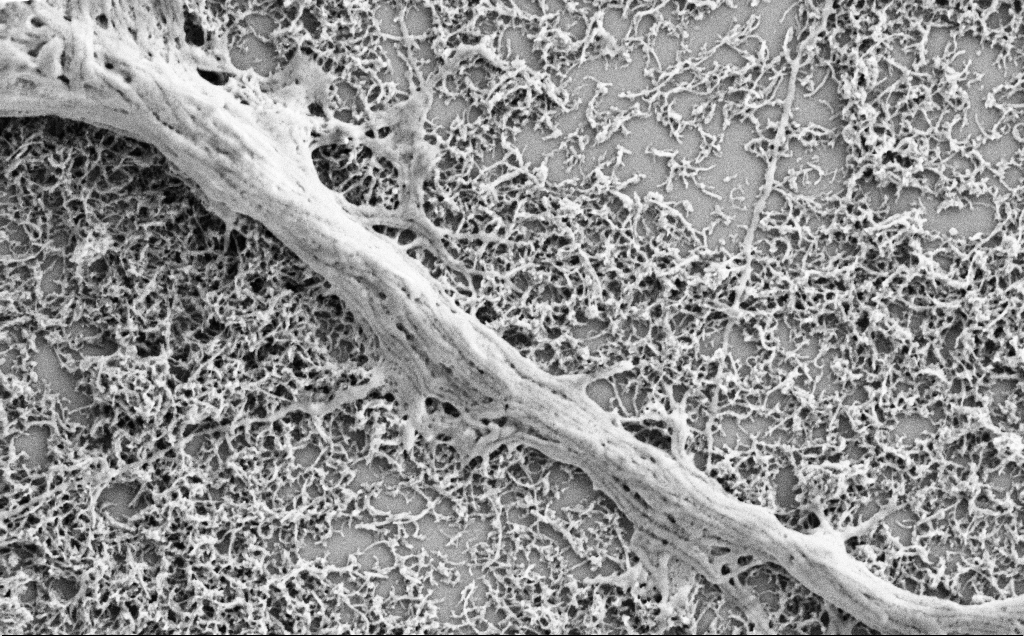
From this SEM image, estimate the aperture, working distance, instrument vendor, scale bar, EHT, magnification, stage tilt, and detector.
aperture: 30 µm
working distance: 7.1 mm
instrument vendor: Zeiss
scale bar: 1000 nm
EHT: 2 kV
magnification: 25 K X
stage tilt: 0°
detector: SE2